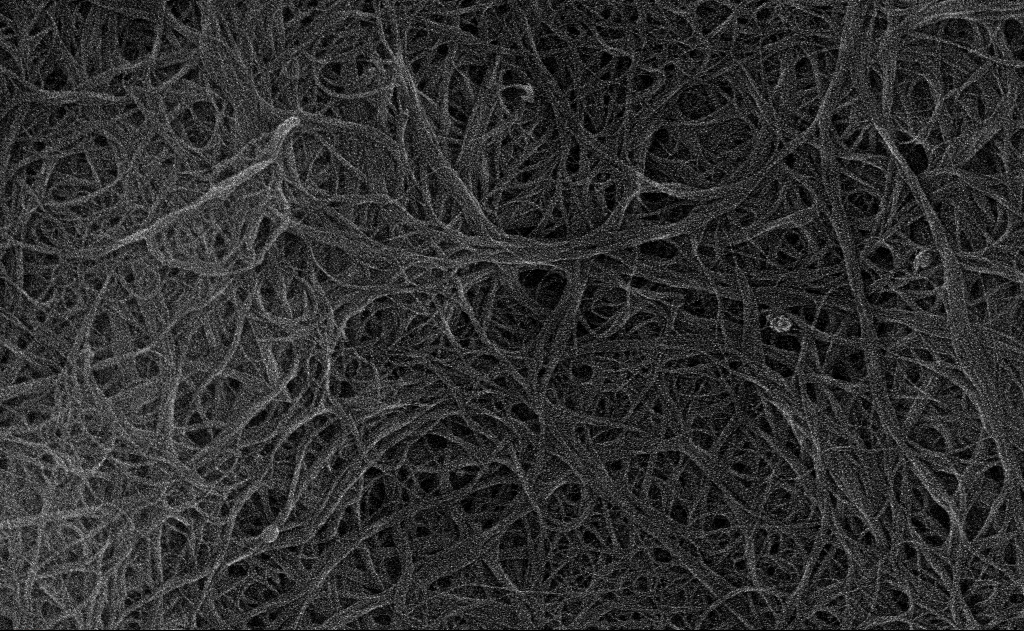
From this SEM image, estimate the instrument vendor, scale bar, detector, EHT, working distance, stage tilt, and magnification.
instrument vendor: Zeiss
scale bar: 200 nm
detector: InLens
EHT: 10 kV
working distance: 3 mm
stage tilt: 0°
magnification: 86.17 K X